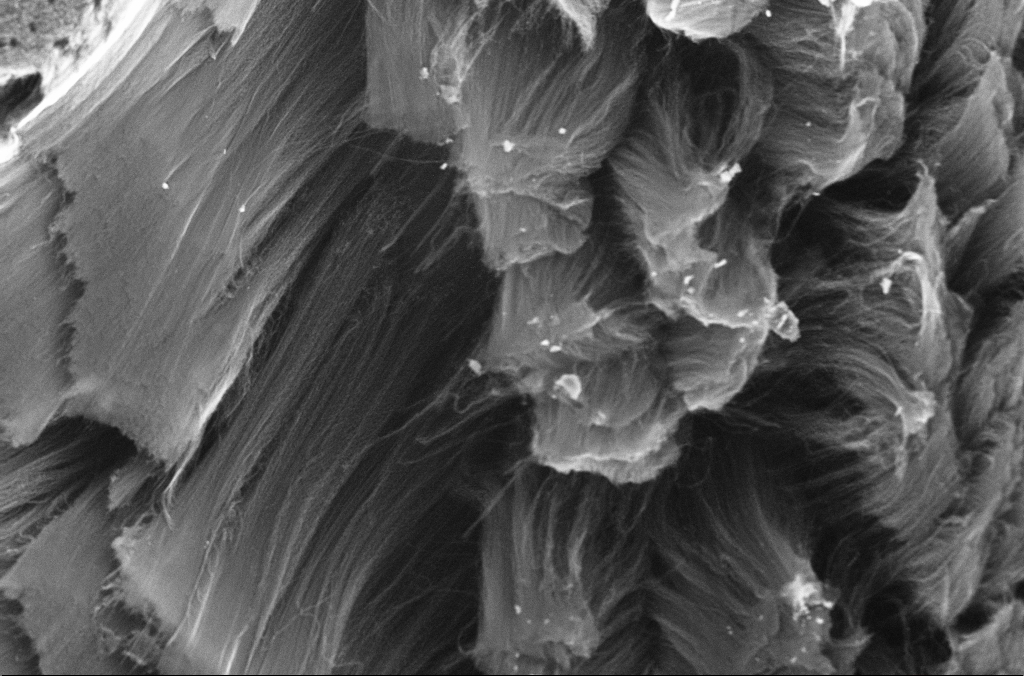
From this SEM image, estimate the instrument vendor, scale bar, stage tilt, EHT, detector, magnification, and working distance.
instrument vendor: Zeiss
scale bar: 10000 nm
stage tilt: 0°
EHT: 10 kV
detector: InLens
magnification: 2.5 K X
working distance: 4 mm